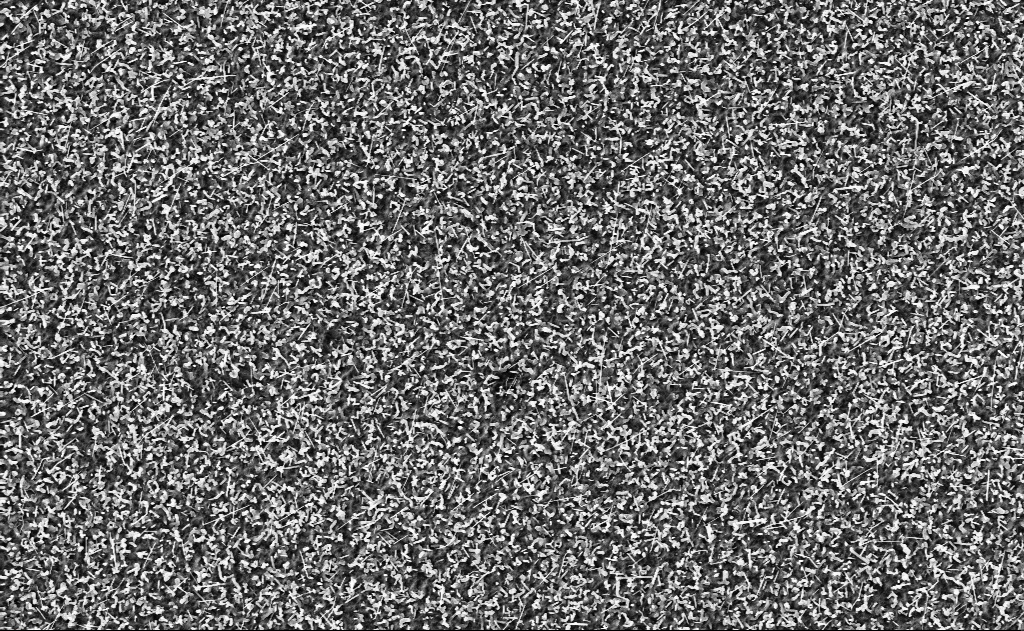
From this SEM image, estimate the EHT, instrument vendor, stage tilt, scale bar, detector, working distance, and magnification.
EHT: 10 kV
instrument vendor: Zeiss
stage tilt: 0°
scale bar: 2000 nm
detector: InLens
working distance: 15 mm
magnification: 10 K X